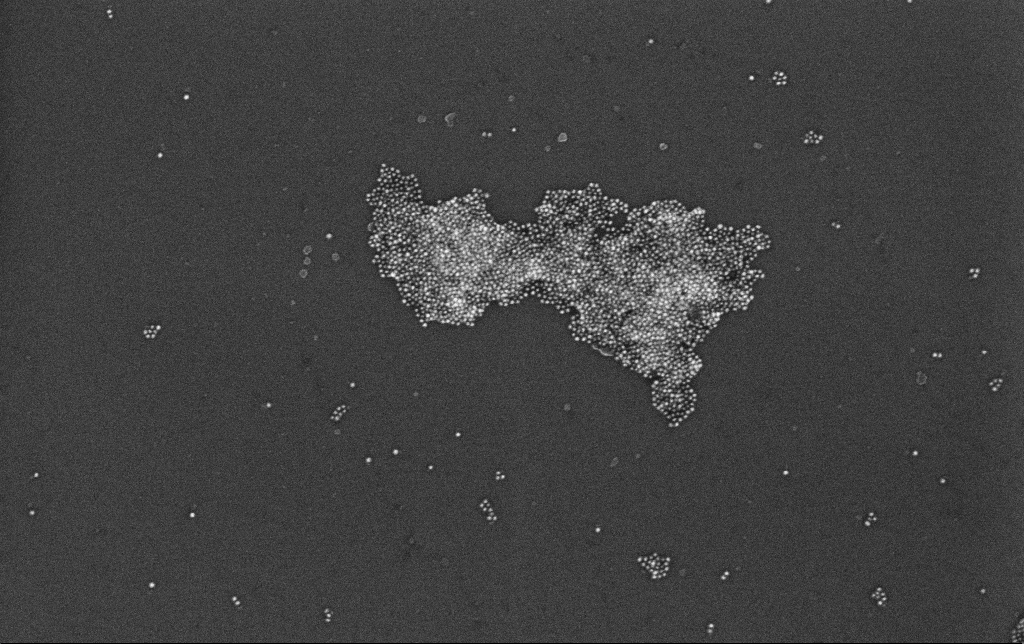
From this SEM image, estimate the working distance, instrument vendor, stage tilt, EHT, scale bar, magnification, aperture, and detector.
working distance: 3.4 mm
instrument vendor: Zeiss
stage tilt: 0°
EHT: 10 kV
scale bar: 200 nm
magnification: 100 K X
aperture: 30 µm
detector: InLens